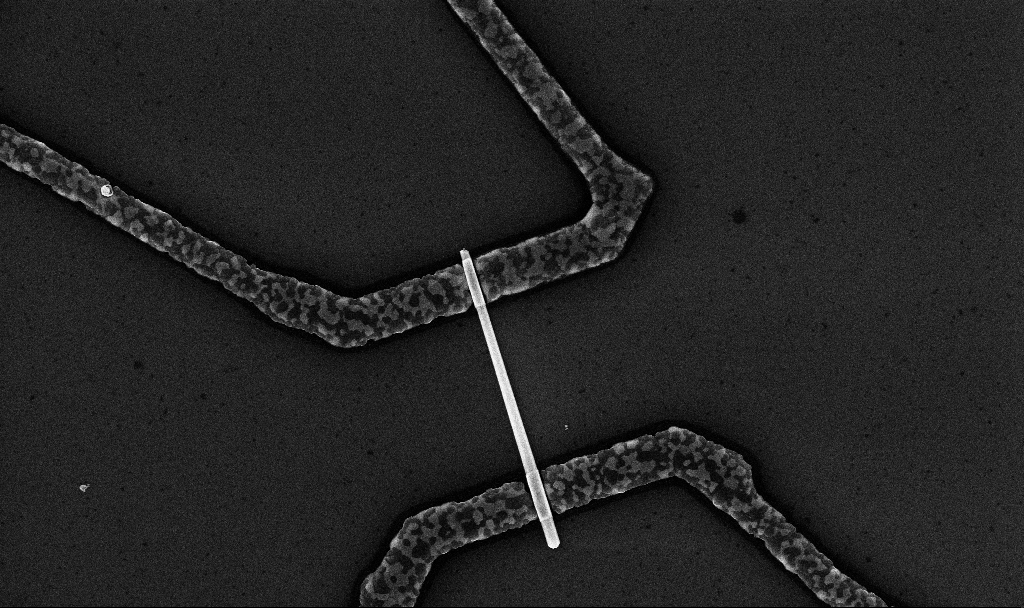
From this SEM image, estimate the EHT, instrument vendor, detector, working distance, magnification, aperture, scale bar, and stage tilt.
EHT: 10 kV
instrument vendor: Zeiss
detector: InLens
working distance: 6.7 mm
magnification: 20 K X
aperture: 30 µm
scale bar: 2000 nm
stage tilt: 0°